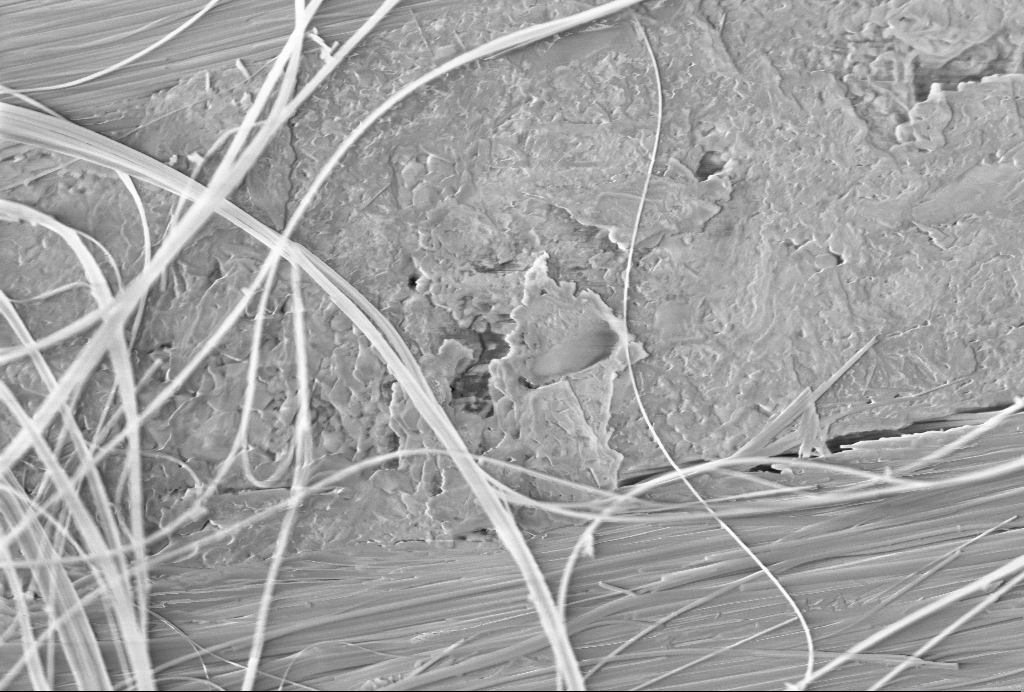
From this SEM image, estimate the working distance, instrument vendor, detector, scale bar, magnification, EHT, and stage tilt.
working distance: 6.7 mm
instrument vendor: Zeiss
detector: InLens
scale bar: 1000 nm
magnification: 26.37 K X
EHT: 3 kV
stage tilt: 45°